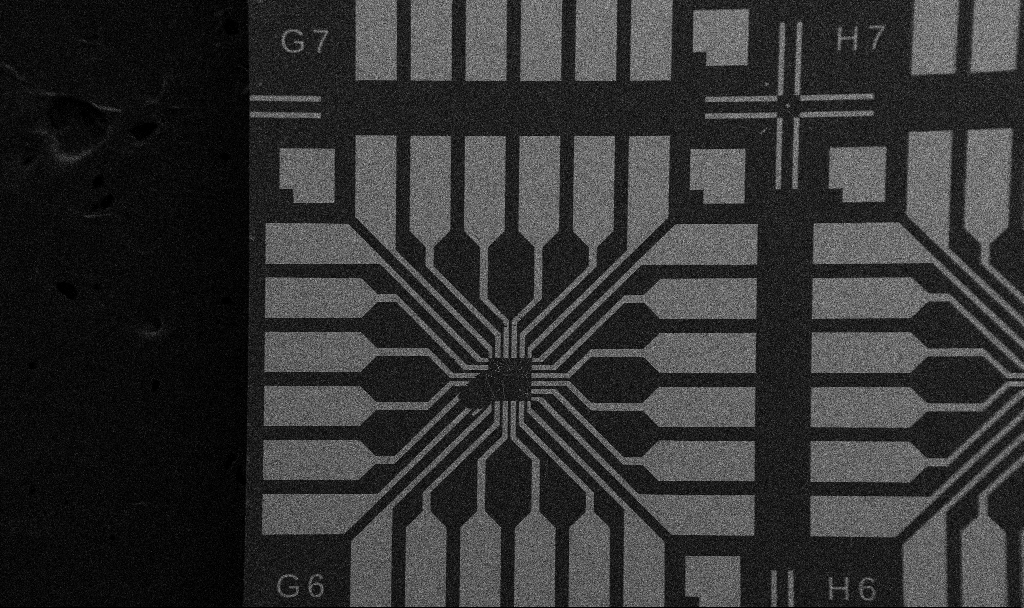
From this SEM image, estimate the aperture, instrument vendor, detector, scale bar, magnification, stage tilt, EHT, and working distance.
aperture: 30 µm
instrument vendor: Zeiss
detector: SE2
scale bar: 200000 nm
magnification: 0.1 K X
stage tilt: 0°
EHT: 5 kV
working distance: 10.7 mm